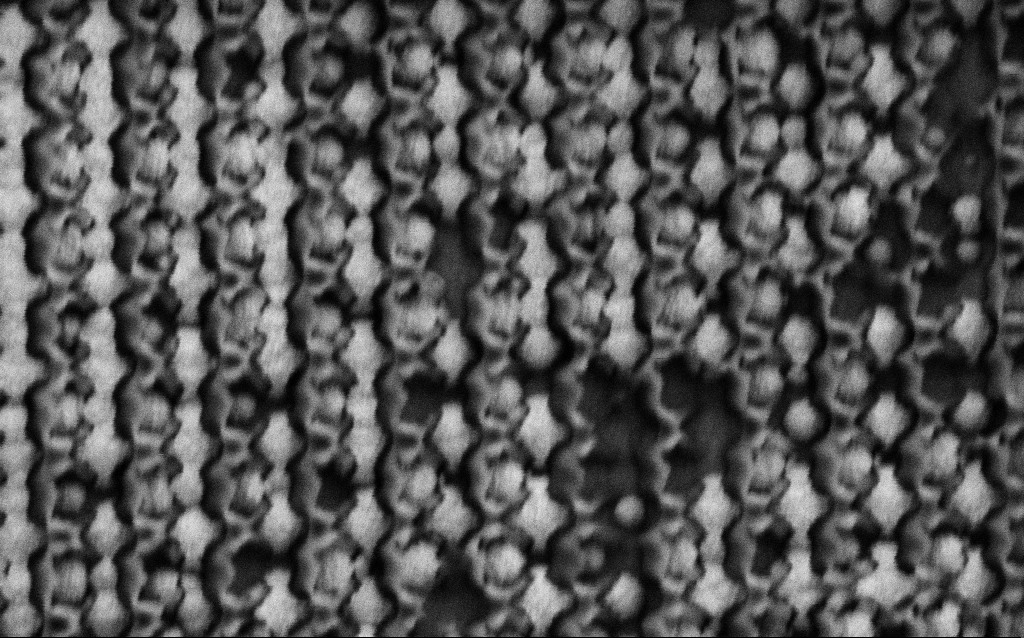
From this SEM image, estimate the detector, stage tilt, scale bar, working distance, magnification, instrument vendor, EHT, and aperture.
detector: SE2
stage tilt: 0°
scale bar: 1000 nm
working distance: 8 mm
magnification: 68.32 K X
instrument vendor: Zeiss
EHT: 1.5 kV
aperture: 30 µm